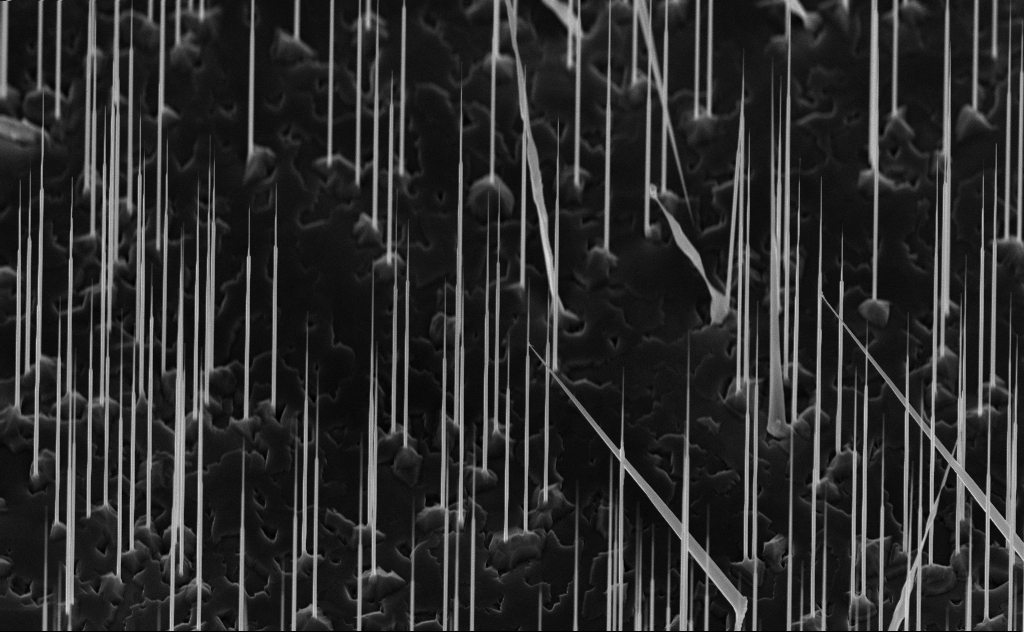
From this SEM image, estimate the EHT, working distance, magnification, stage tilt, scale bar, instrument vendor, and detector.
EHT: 10 kV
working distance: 6 mm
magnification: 10 K X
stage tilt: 45°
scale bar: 2000 nm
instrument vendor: Zeiss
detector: InLens